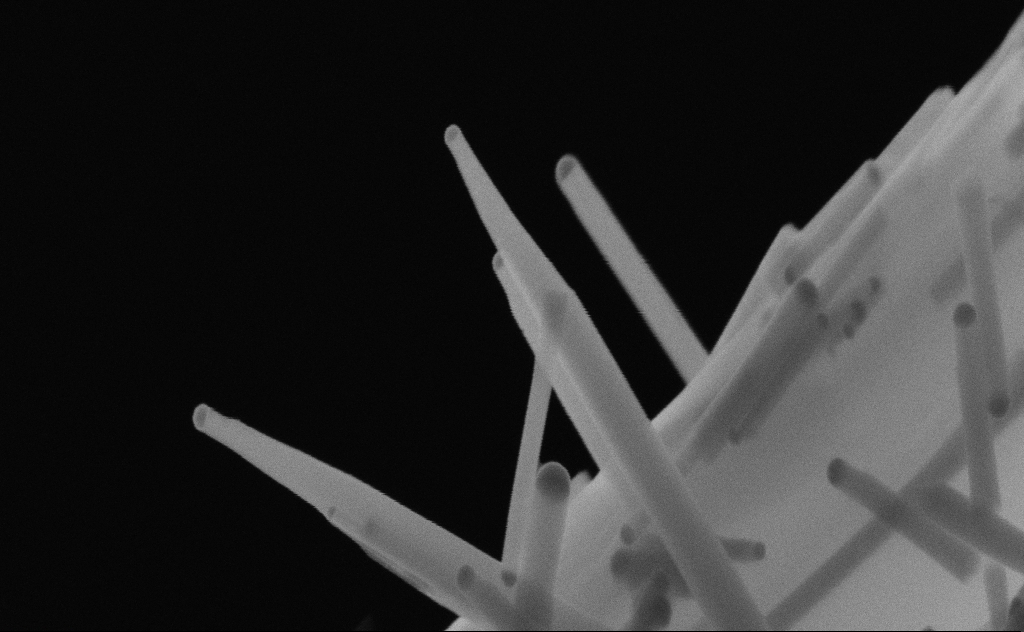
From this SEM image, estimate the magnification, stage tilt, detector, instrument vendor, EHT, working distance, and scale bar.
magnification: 151.23 K X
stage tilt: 0°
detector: SE2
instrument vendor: Zeiss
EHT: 20 kV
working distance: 9 mm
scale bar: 200 nm